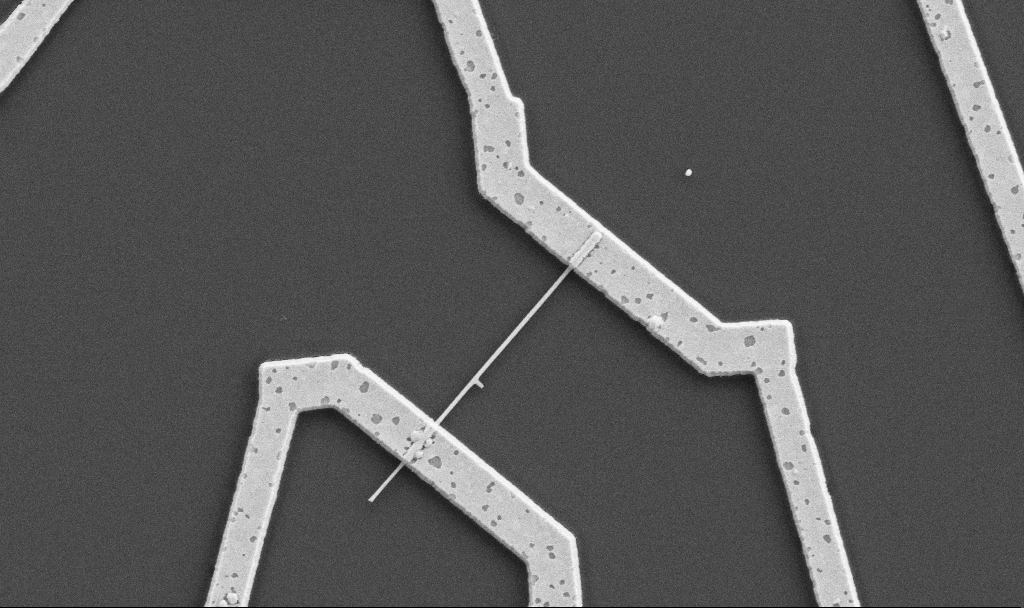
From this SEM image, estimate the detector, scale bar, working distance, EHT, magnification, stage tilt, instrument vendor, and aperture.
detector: SE2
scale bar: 1000 nm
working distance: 10.7 mm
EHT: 5 kV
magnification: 20 K X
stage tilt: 0°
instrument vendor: Zeiss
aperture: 30 µm